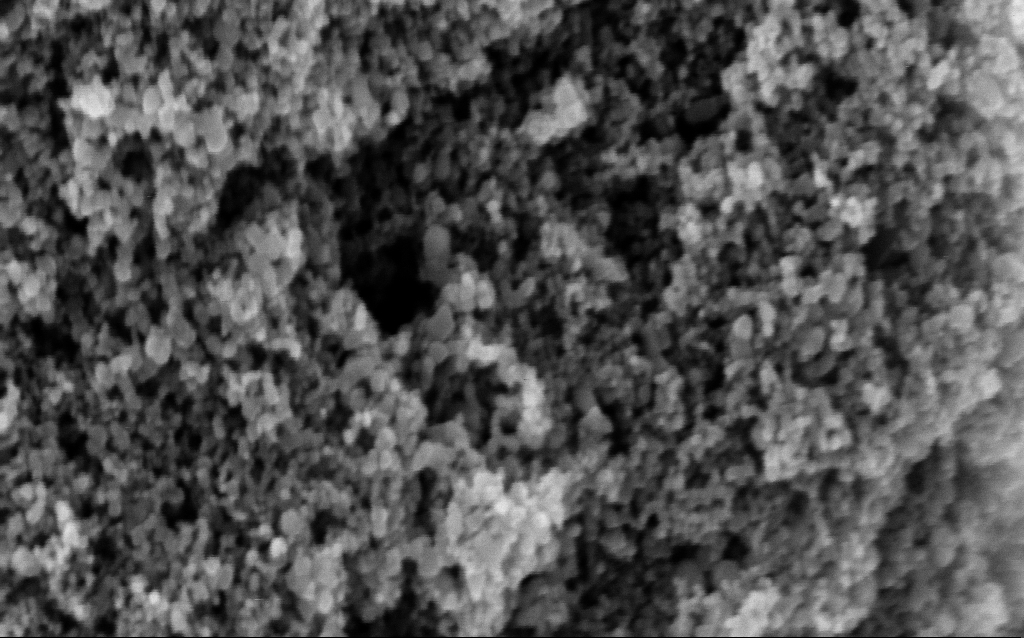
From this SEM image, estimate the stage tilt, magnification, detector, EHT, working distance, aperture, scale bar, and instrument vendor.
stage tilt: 0°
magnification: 142.44 K X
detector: InLens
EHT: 5 kV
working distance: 9.6 mm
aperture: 30 µm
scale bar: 200 nm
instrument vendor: Zeiss